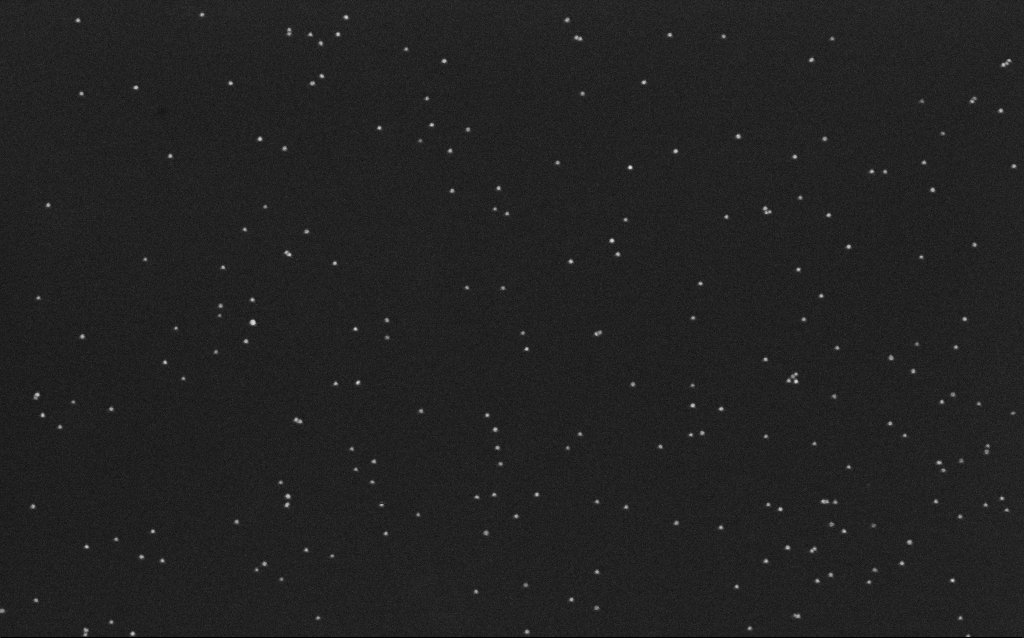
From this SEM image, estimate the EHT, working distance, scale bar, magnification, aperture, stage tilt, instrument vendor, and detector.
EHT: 10 kV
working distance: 6.5 mm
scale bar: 200 nm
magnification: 100 K X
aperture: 30 µm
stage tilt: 0°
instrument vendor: Zeiss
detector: InLens